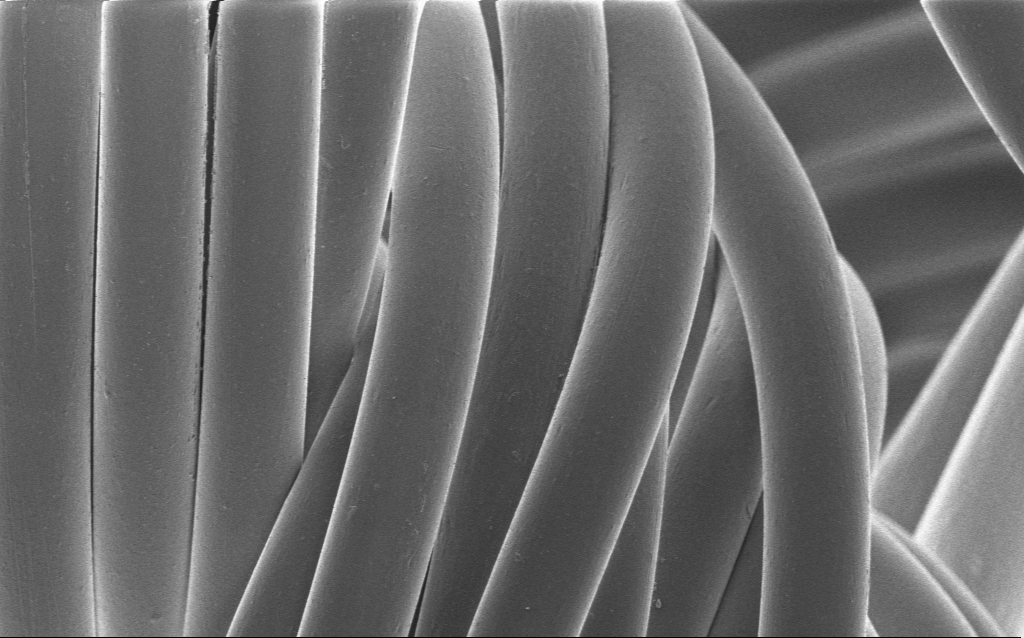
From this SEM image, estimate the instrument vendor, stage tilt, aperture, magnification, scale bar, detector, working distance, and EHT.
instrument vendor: Zeiss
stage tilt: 0°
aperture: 30 µm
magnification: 1.63 K X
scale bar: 20000 nm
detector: InLens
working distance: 4 mm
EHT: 1 kV